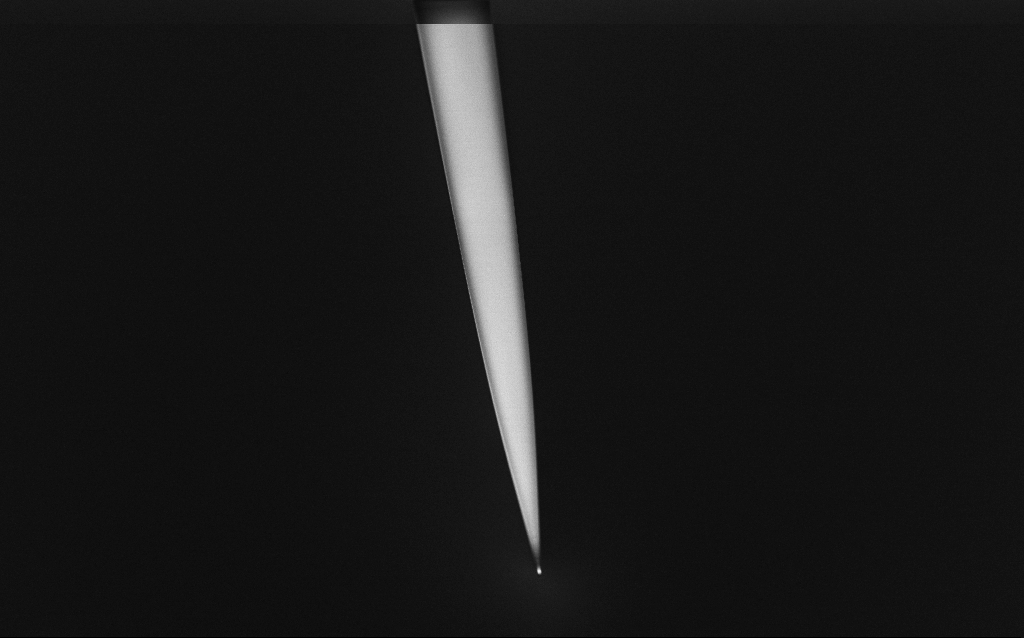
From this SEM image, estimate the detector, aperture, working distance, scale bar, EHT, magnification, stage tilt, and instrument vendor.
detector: InLens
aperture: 30 µm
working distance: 6 mm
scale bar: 20000 nm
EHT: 1 kV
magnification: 1 K X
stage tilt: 45°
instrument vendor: Zeiss